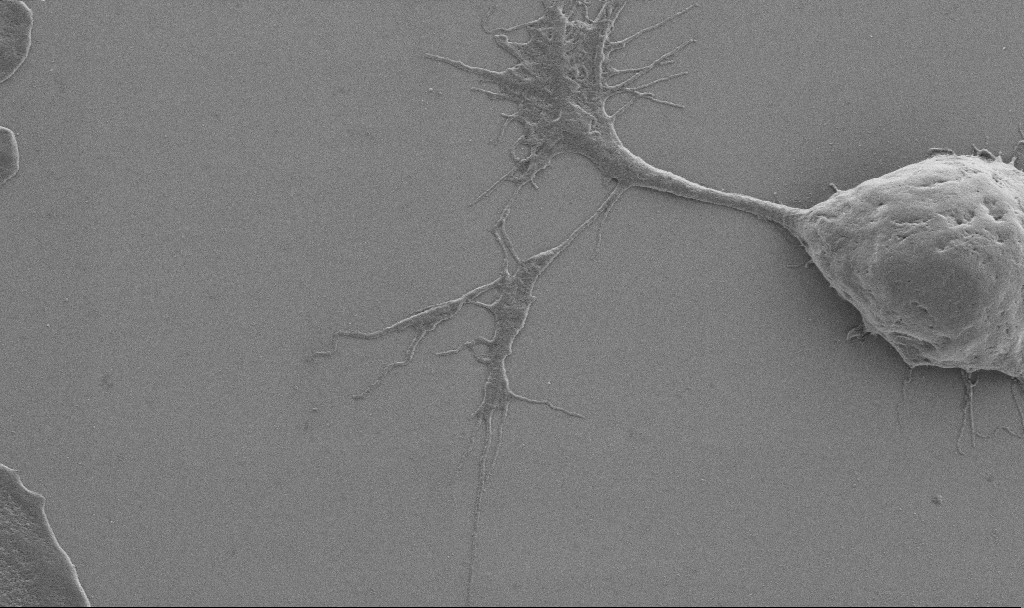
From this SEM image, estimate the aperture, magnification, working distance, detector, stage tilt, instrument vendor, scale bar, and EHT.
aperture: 30 µm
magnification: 10 K X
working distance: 6.9 mm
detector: SE2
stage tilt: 0°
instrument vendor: Zeiss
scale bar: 2000 nm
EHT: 0.9 kV